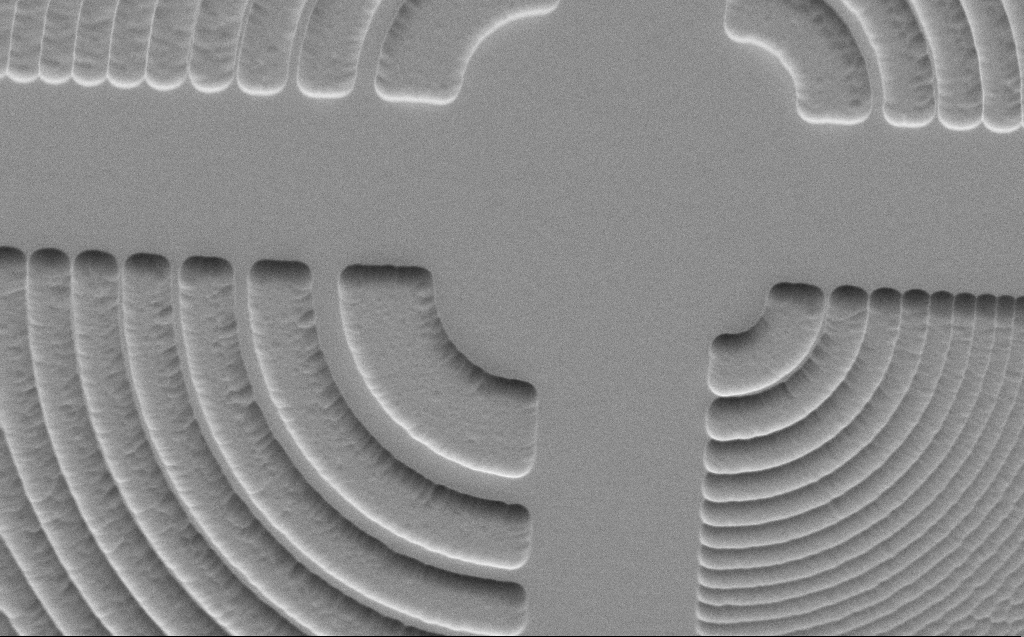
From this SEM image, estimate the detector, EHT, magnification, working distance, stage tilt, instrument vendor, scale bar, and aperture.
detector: SE2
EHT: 3 kV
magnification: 6.98 K X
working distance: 5 mm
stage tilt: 45°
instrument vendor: Zeiss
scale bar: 10000 nm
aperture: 30 µm